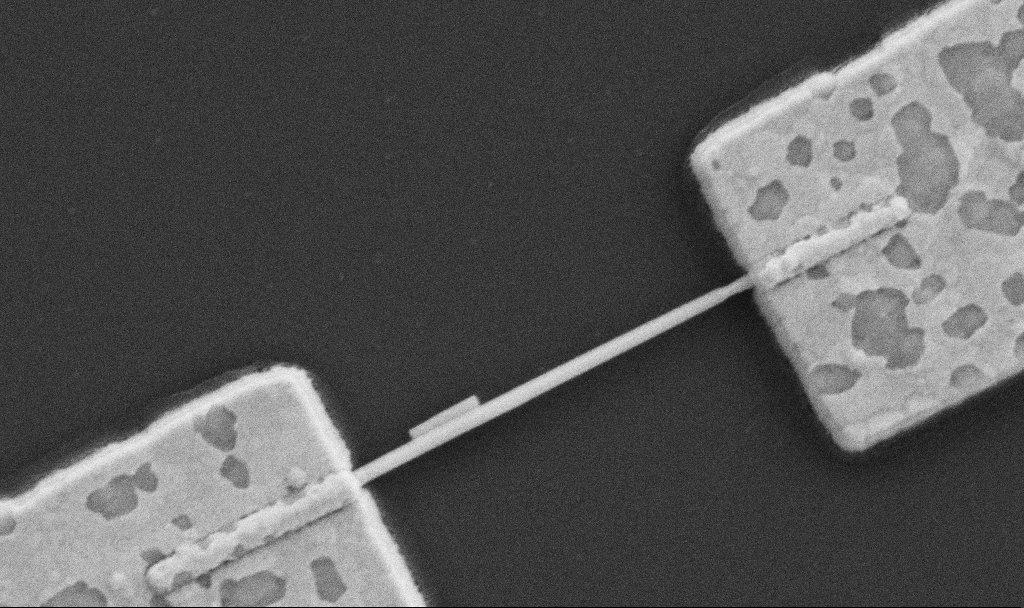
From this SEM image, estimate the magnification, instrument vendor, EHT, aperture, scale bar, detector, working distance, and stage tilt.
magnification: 60 K X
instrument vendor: Zeiss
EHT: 5 kV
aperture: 30 µm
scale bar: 1000 nm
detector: SE2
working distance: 10.7 mm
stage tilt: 0°